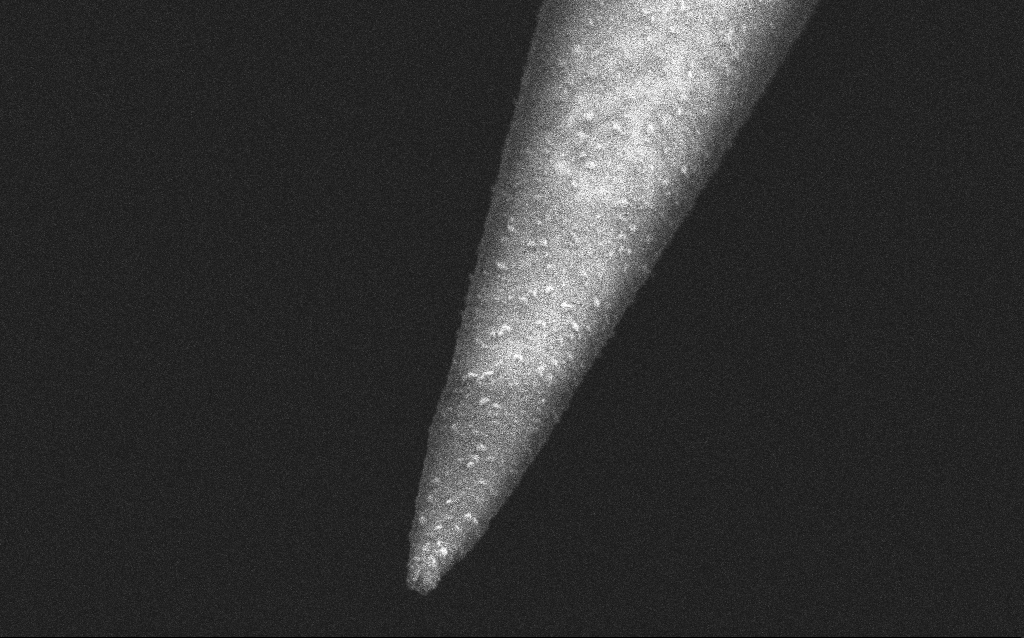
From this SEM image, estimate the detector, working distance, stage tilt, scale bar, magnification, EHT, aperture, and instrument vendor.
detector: InLens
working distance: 5 mm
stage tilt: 45°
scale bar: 200 nm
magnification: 100 K X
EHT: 2.5 kV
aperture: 30 µm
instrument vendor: Zeiss